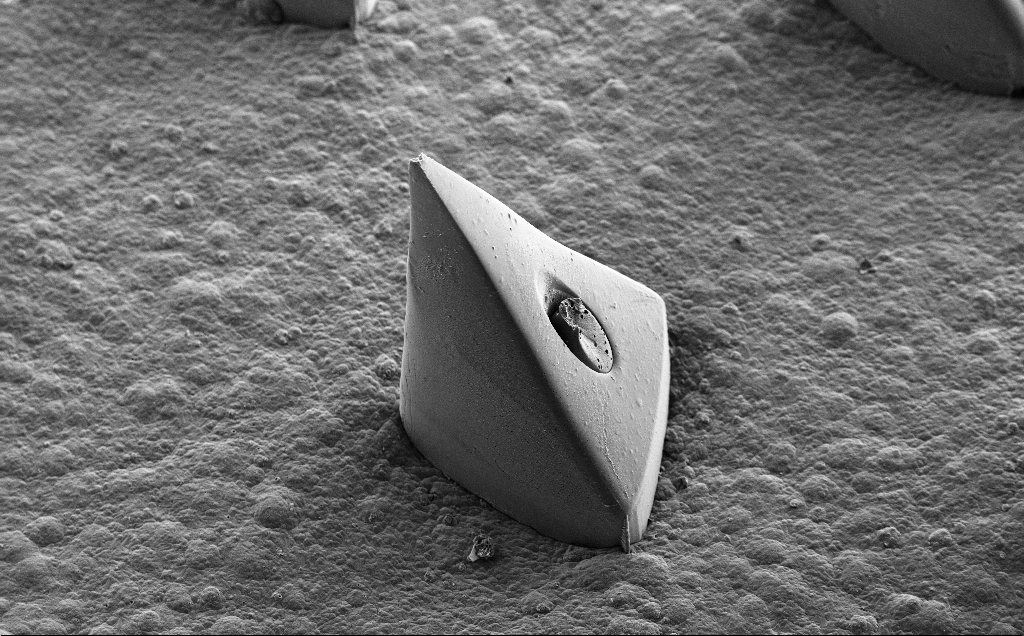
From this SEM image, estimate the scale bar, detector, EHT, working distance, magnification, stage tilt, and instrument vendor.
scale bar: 200000 nm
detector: SE2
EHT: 10 kV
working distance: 10 mm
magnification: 0.175 K X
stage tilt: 35.3°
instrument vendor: Zeiss